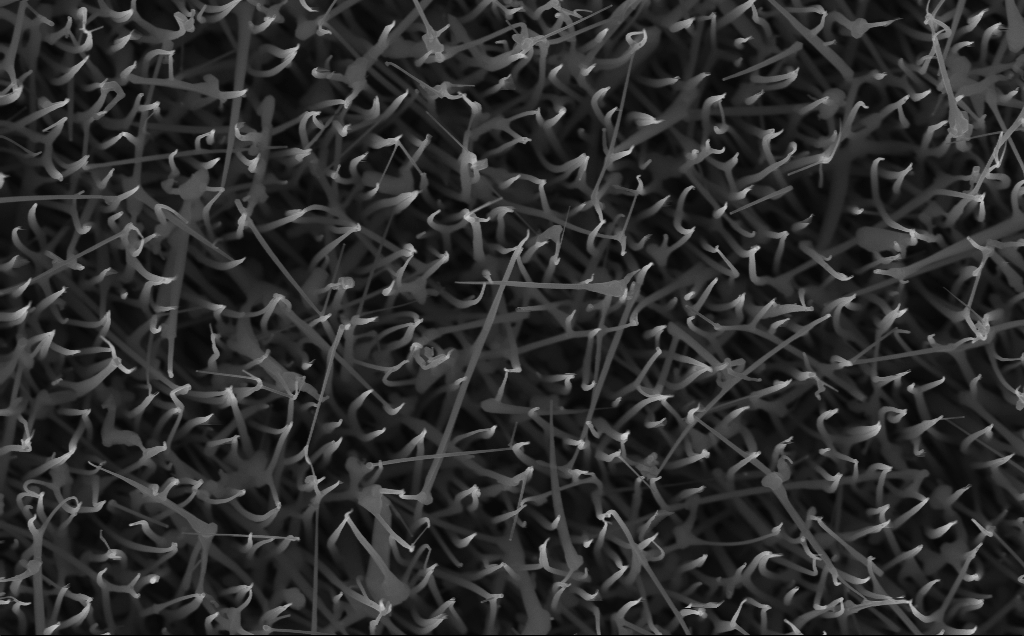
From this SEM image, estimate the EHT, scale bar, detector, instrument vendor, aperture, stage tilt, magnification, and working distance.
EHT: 10 kV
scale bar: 1000 nm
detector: InLens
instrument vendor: Zeiss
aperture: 30 µm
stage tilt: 0°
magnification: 40 K X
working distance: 4 mm